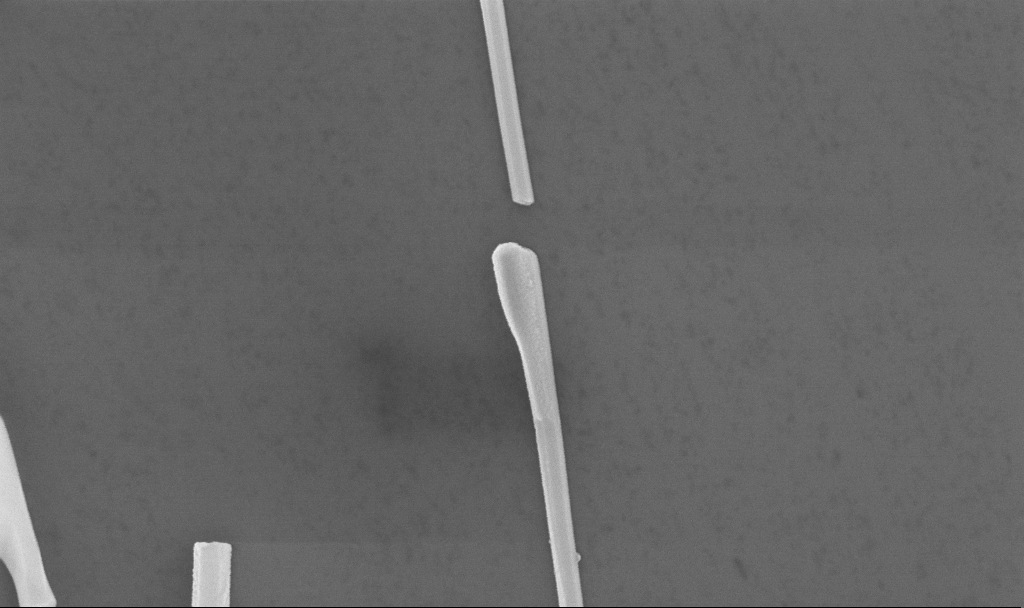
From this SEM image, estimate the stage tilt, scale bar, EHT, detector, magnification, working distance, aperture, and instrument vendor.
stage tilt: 0°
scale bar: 1000 nm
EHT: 10 kV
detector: InLens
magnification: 72.16 K X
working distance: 8.7 mm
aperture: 30 µm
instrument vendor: Zeiss